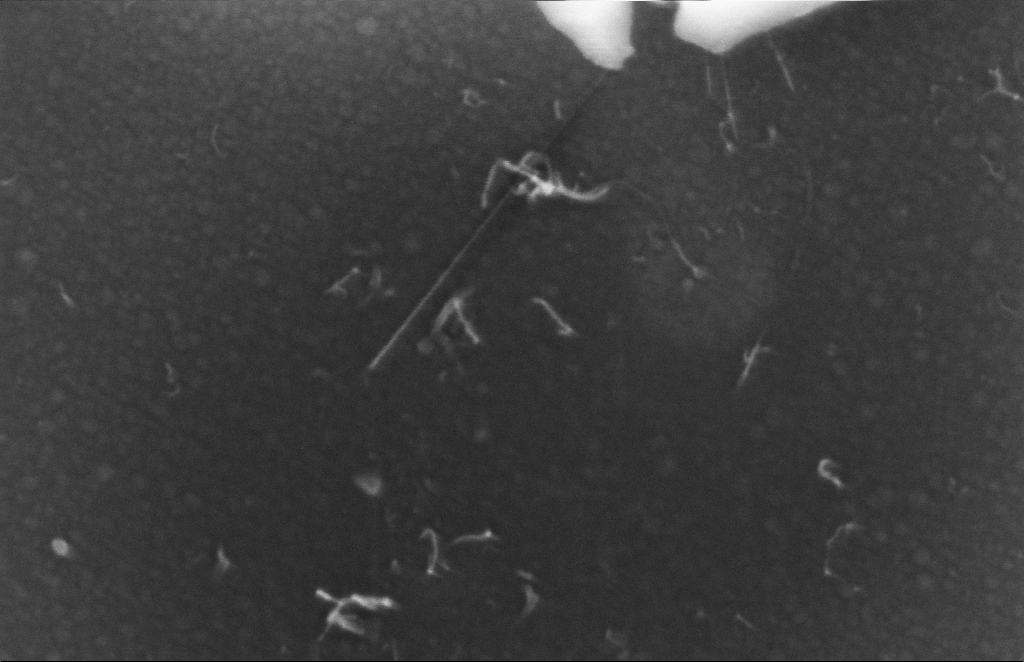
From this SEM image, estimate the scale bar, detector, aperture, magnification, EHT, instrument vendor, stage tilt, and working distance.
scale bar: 100 nm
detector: InLens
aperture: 30 µm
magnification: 337.46 K X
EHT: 5 kV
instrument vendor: Zeiss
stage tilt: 0°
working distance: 5 mm